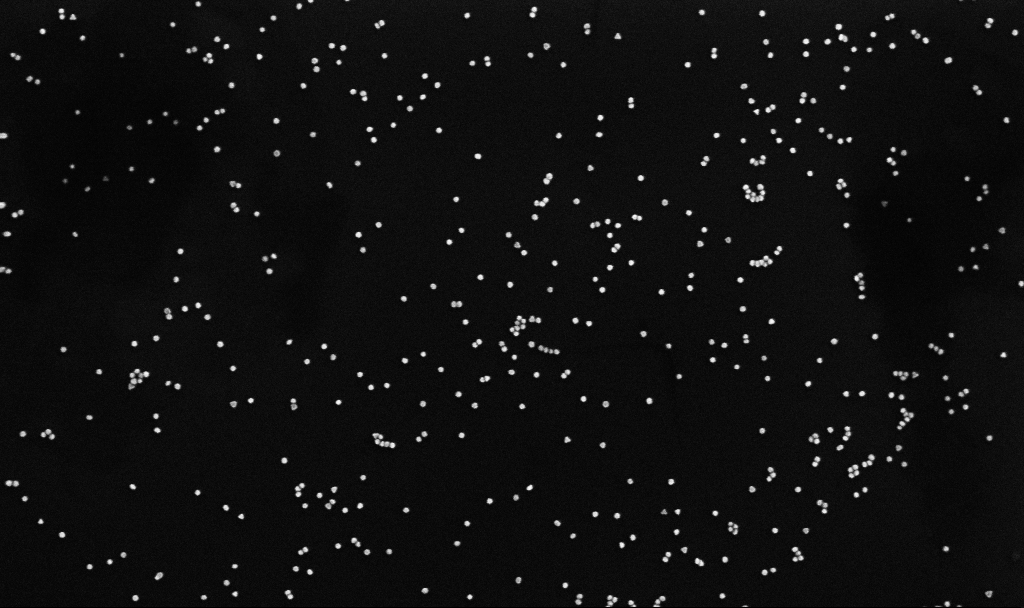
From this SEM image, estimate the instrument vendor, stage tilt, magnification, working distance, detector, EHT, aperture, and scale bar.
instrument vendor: Zeiss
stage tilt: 0°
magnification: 93.53 K X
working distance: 3.2 mm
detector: InLens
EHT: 8 kV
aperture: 30 µm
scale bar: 200 nm